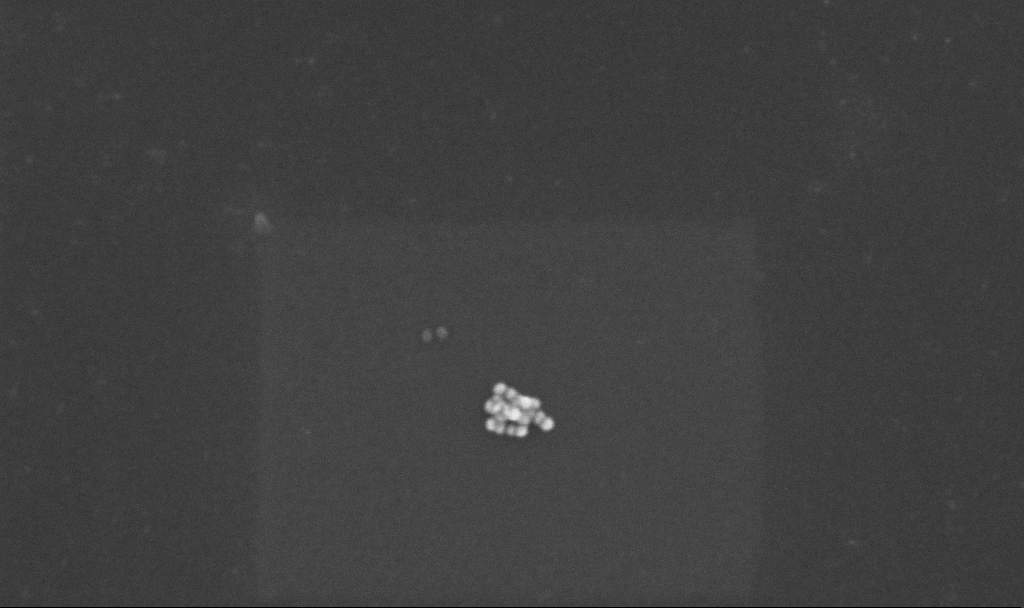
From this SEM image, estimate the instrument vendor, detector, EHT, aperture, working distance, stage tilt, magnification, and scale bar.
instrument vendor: Zeiss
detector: InLens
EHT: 10 kV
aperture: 30 µm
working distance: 3.2 mm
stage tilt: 0°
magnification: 217.47 K X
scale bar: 100 nm